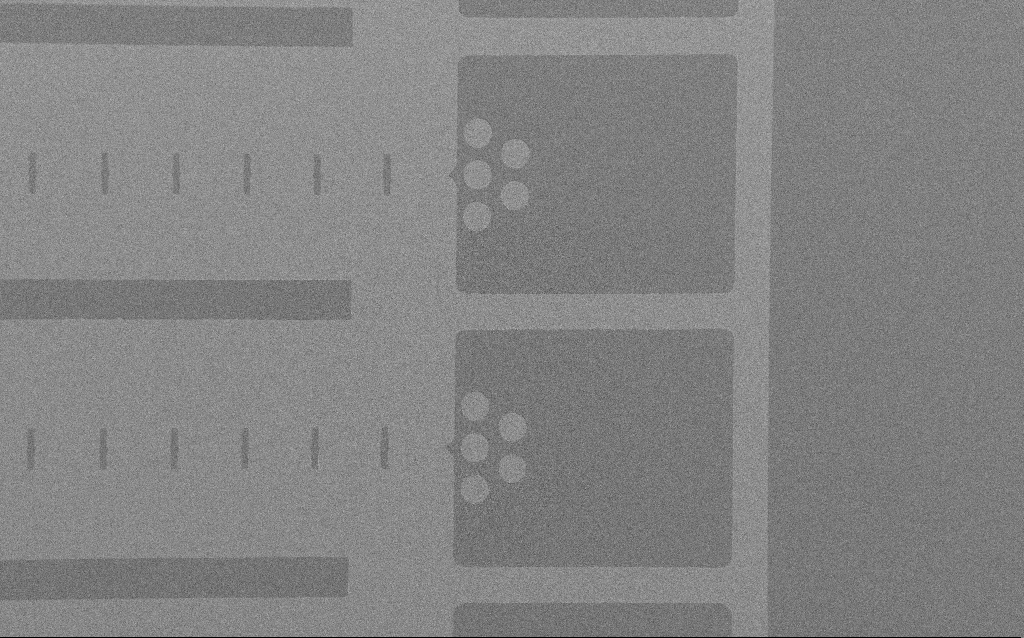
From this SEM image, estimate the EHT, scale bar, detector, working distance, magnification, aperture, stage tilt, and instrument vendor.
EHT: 2 kV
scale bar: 200000 nm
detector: SE2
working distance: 5 mm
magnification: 0.259 K X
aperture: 30 µm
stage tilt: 0°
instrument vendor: Zeiss